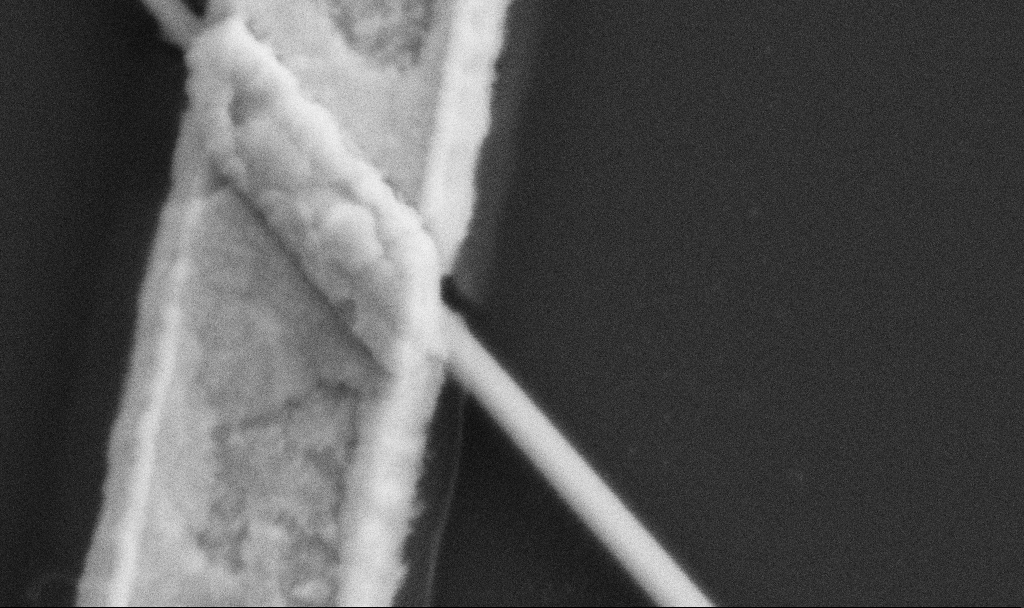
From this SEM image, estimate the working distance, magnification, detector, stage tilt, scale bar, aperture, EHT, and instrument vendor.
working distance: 10.7 mm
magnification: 150 K X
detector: SE2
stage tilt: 0°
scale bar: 100 nm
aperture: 30 µm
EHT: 5 kV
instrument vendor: Zeiss